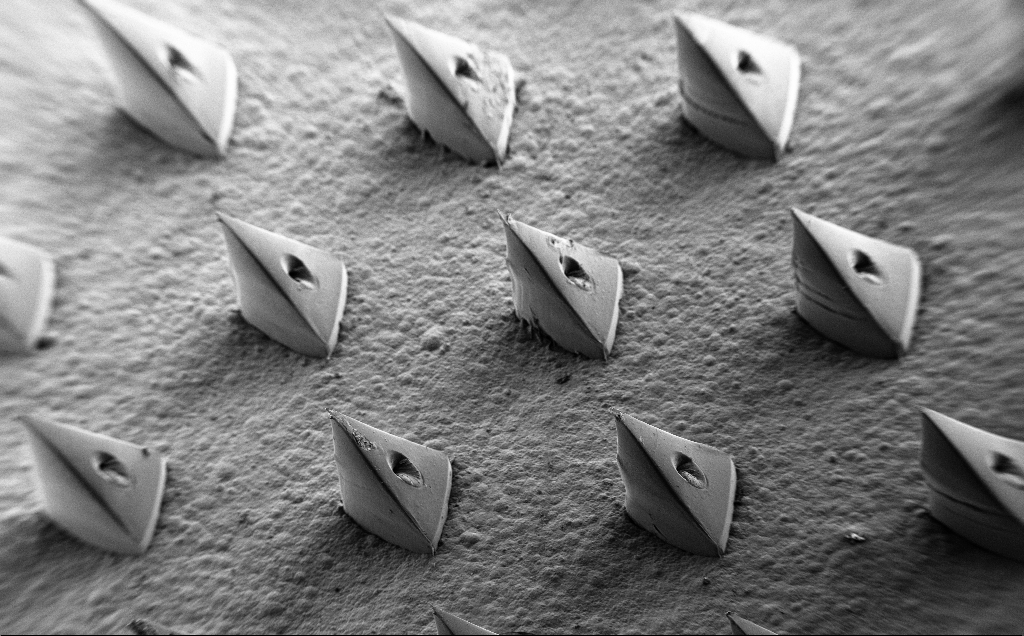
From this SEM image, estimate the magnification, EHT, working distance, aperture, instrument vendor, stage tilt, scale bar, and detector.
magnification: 0.073 K X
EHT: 5 kV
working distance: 11 mm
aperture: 30 µm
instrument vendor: Zeiss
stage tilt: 35°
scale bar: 200000 nm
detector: SE2